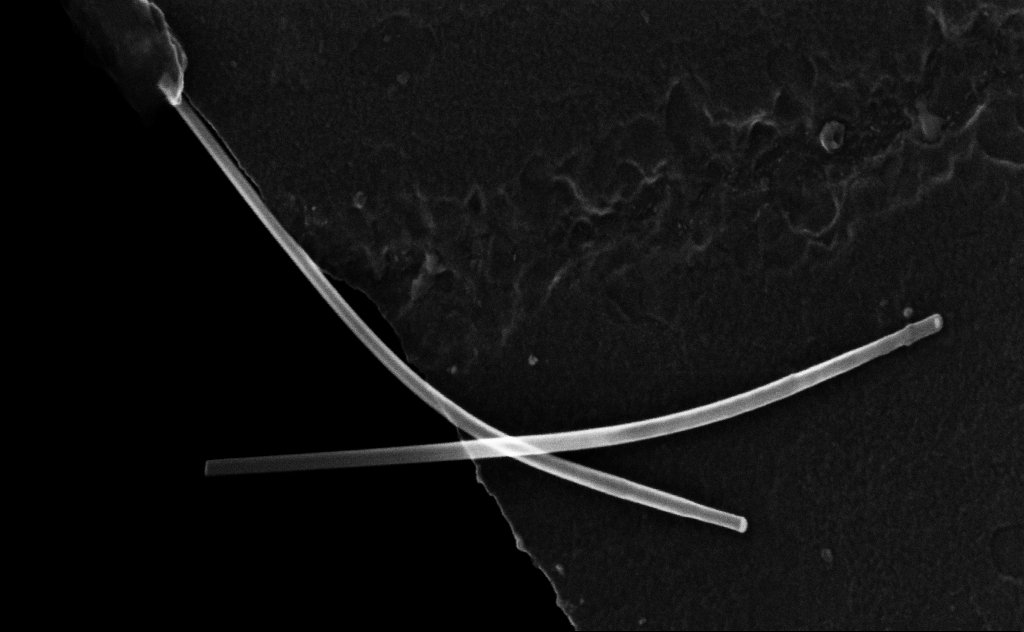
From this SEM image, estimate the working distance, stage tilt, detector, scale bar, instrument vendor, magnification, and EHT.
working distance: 8 mm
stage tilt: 0°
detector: InLens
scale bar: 100 nm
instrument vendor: Zeiss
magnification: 161.84 K X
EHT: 20 kV